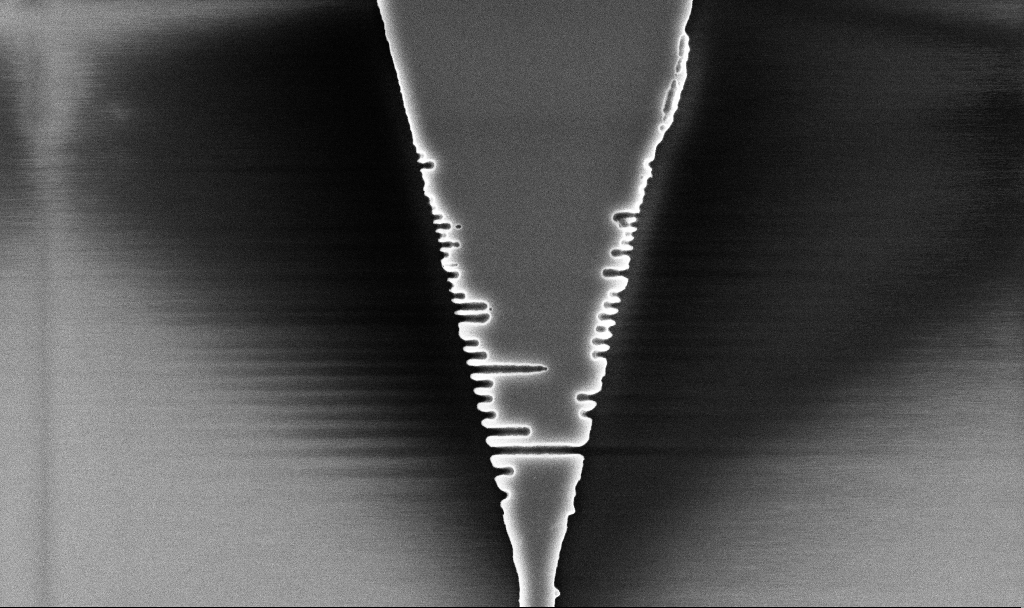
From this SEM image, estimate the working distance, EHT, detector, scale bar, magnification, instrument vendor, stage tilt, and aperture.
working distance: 5.2 mm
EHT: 5 kV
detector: InLens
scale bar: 2000 nm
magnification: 25.89 K X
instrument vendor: Zeiss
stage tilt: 0°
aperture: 30 µm